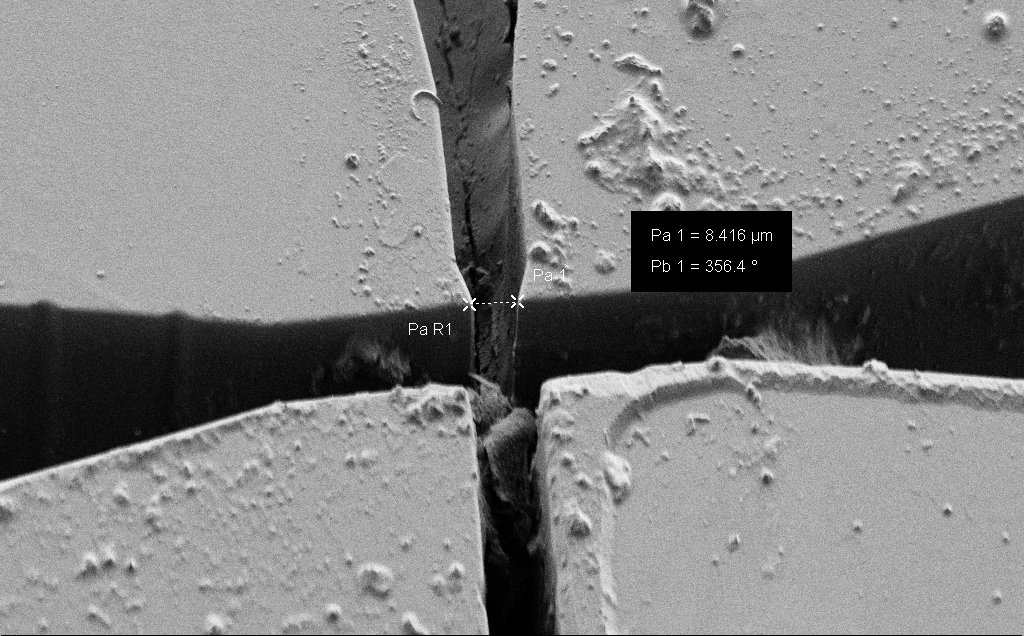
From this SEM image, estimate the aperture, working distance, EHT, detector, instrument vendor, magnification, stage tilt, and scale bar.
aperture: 30 µm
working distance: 6 mm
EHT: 1 kV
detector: SE2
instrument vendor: Zeiss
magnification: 2.1 K X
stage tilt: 45°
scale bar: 20000 nm